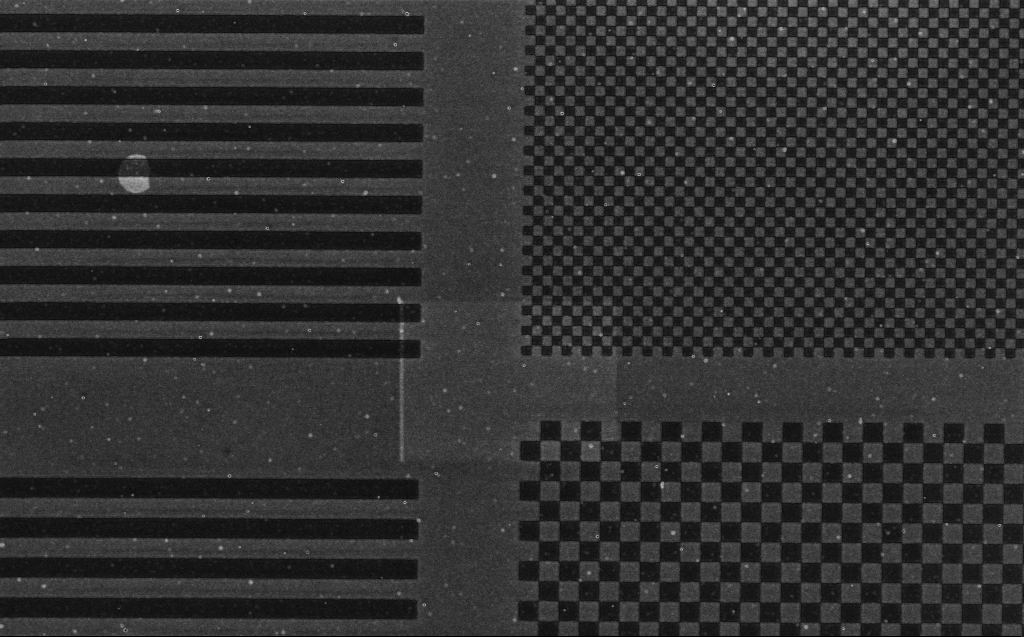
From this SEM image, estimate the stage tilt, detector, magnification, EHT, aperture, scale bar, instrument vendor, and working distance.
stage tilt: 0°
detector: InLens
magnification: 7.41 K X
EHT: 1.2 kV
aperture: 30 µm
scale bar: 2000 nm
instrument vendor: Zeiss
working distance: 5 mm